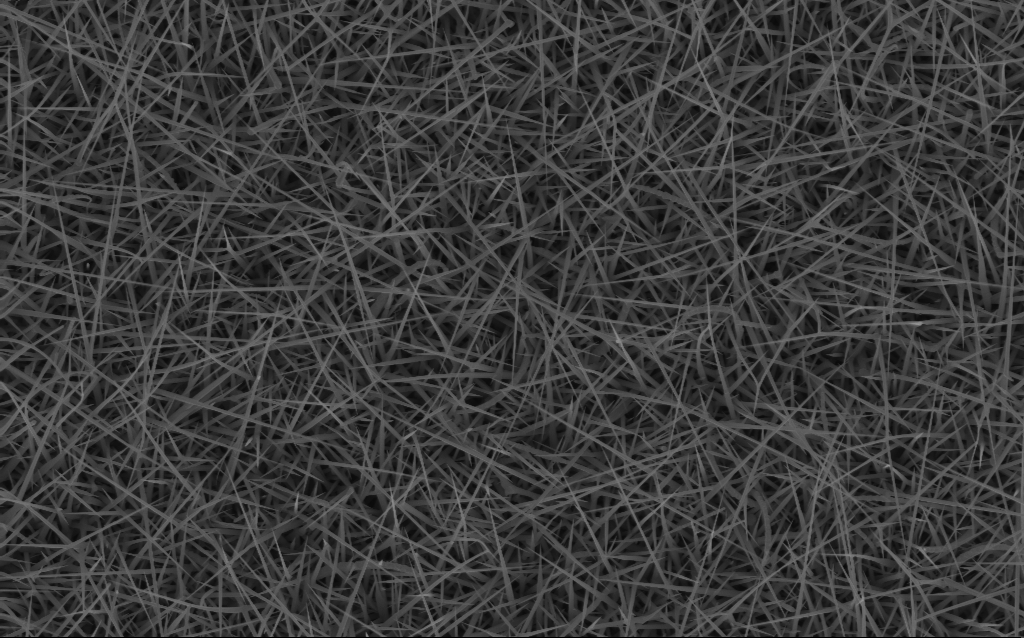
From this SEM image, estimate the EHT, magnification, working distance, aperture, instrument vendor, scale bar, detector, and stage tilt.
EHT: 10 kV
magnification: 10 K X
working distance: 7 mm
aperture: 30 µm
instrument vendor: Zeiss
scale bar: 2000 nm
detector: InLens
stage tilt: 0°